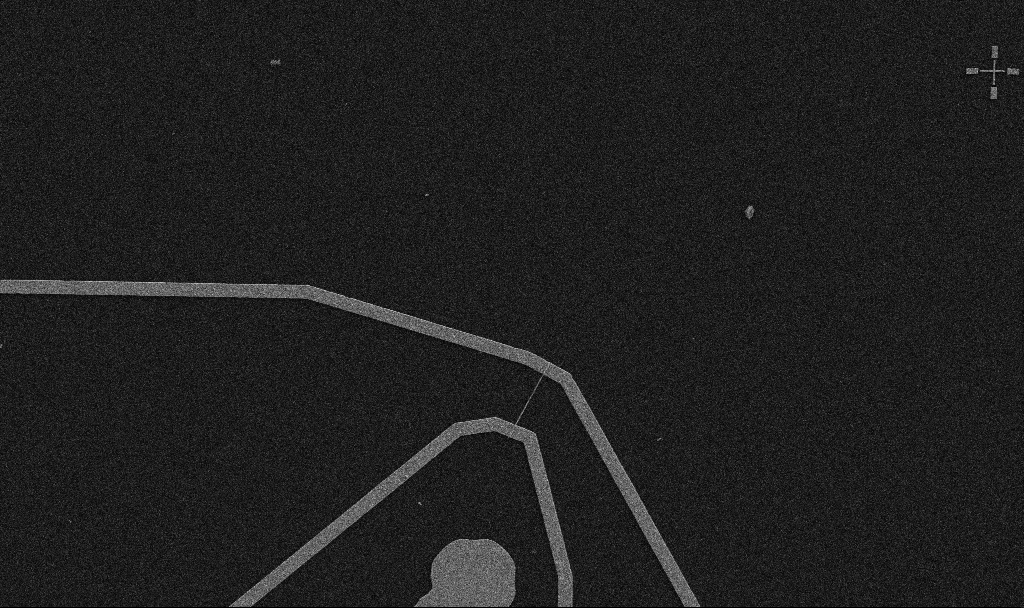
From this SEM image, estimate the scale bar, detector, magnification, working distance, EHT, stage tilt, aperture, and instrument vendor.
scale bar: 10000 nm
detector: SE2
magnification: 5 K X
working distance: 10.7 mm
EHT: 5 kV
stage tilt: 0°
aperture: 30 µm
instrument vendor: Zeiss